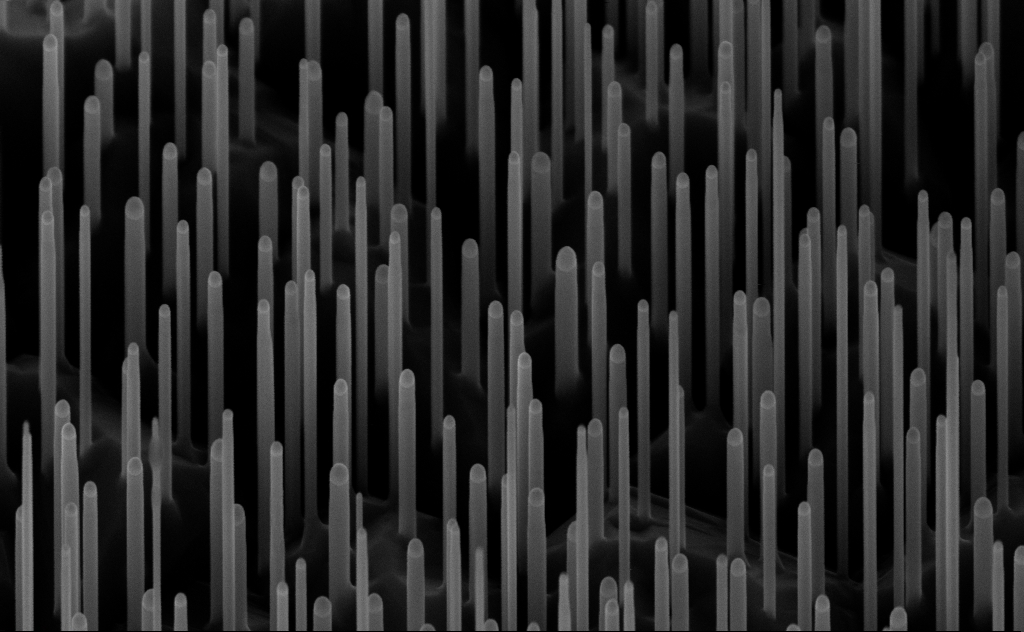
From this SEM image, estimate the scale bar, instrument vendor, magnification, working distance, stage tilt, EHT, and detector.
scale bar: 200 nm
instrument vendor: Zeiss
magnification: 80 K X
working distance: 7 mm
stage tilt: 45°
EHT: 10 kV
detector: InLens